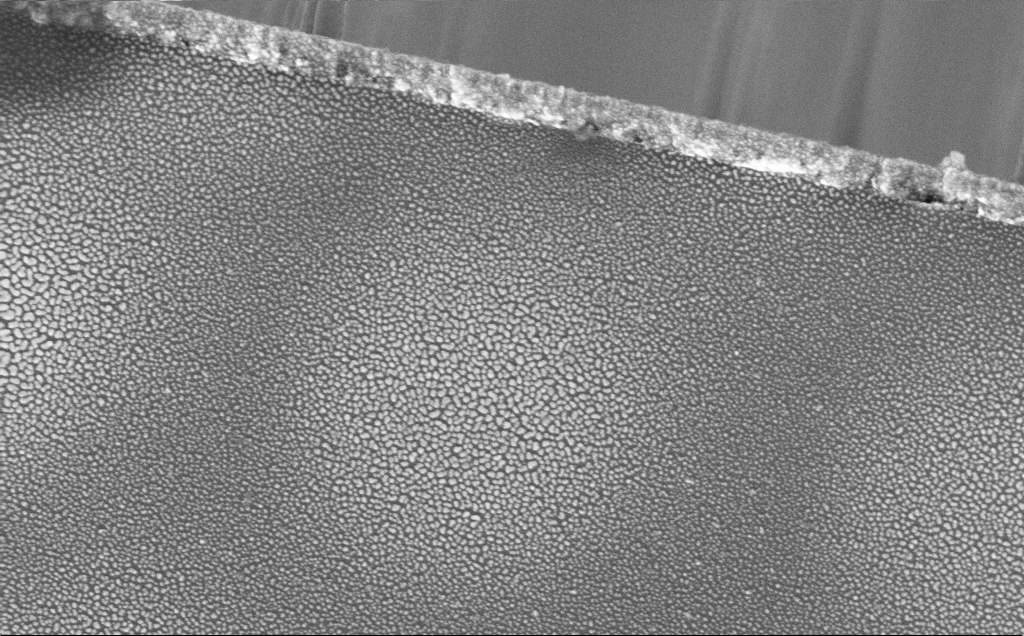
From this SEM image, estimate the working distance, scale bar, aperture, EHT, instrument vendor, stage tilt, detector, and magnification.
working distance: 11 mm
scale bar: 1000 nm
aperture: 30 µm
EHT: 5 kV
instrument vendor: Zeiss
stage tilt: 0°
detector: InLens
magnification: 62.56 K X